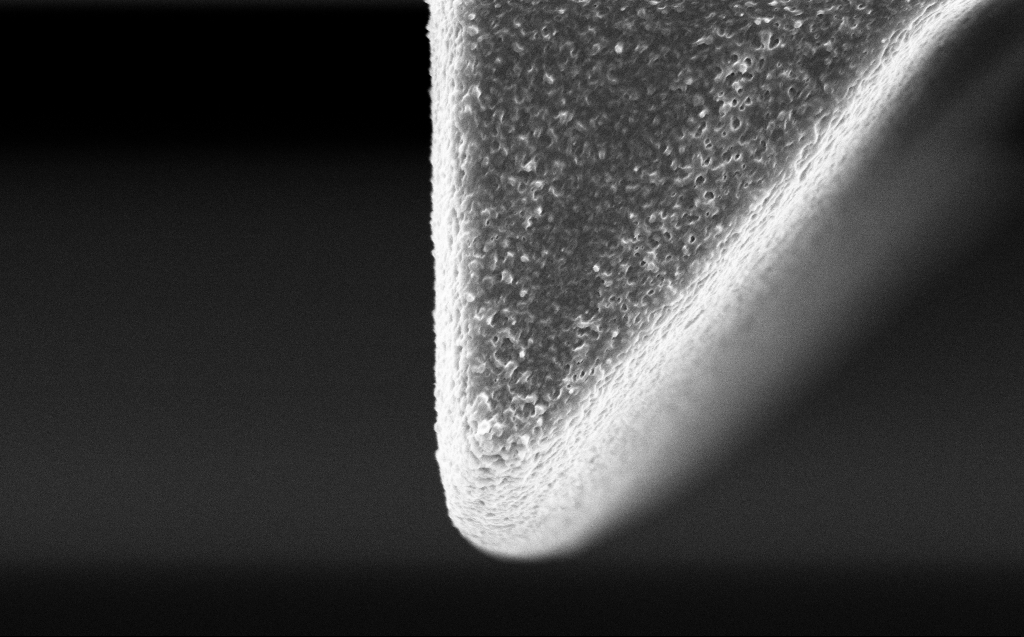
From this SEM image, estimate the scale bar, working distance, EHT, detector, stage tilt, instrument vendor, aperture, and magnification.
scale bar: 2000 nm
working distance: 7 mm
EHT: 5 kV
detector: InLens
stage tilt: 0°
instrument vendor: Zeiss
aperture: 30 µm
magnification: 25.1 K X